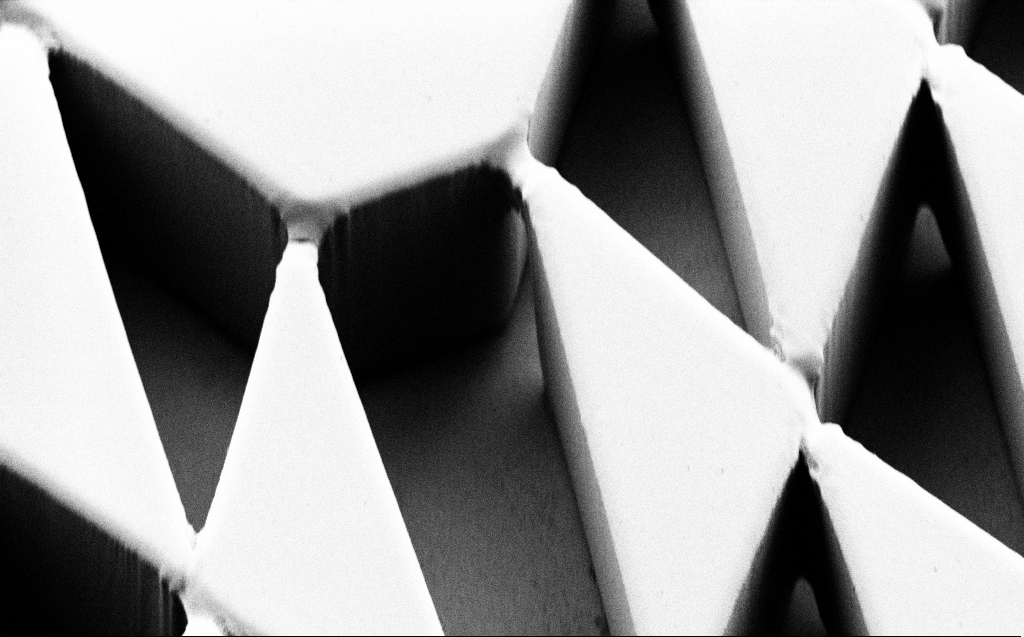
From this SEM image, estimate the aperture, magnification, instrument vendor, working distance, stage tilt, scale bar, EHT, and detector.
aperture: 30 µm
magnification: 2.26 K X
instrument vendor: Zeiss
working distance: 6 mm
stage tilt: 45°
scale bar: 20000 nm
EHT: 1 kV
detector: SE2